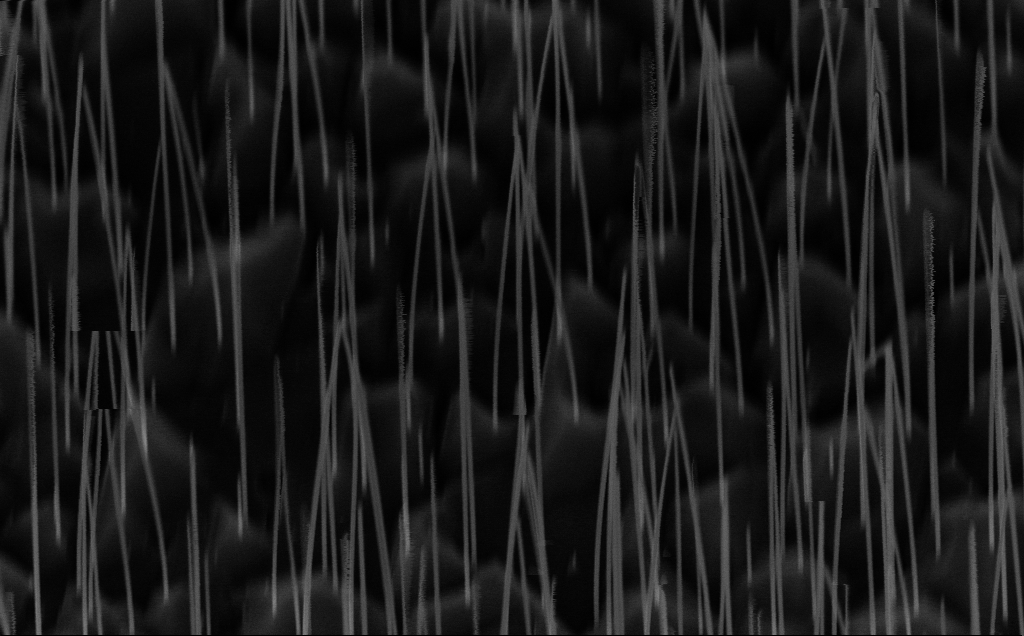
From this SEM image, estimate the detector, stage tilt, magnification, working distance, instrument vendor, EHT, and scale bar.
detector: InLens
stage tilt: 44.9°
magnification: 51.21 K X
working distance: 7 mm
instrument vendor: Zeiss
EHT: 10 kV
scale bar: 1000 nm